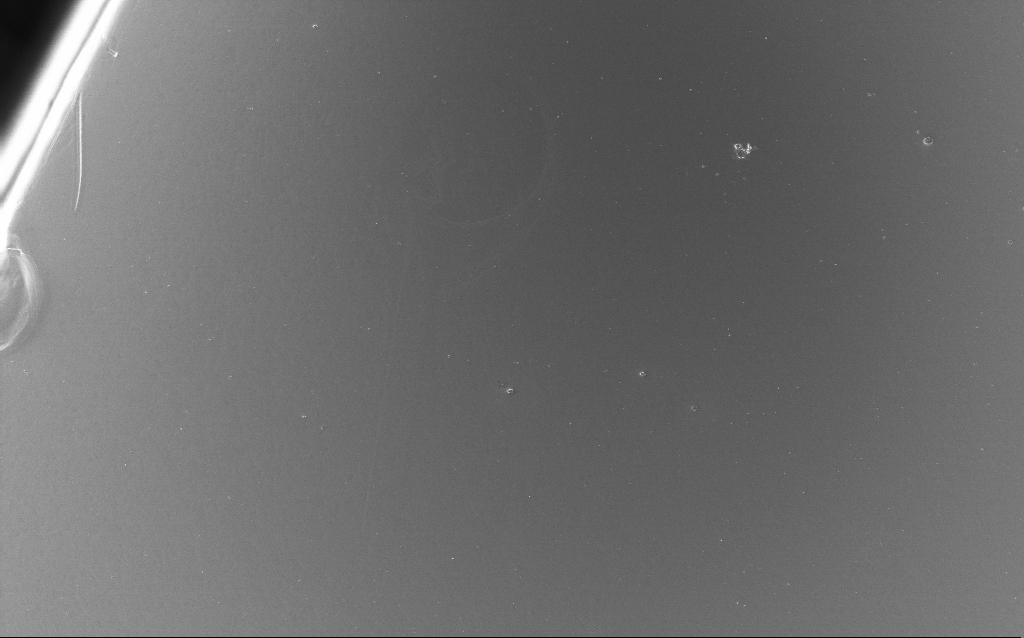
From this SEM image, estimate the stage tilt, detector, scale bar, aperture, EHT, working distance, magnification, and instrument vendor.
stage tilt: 0°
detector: InLens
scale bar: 100000 nm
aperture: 30 µm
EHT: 5 kV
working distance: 2 mm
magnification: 0.662 K X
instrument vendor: Zeiss